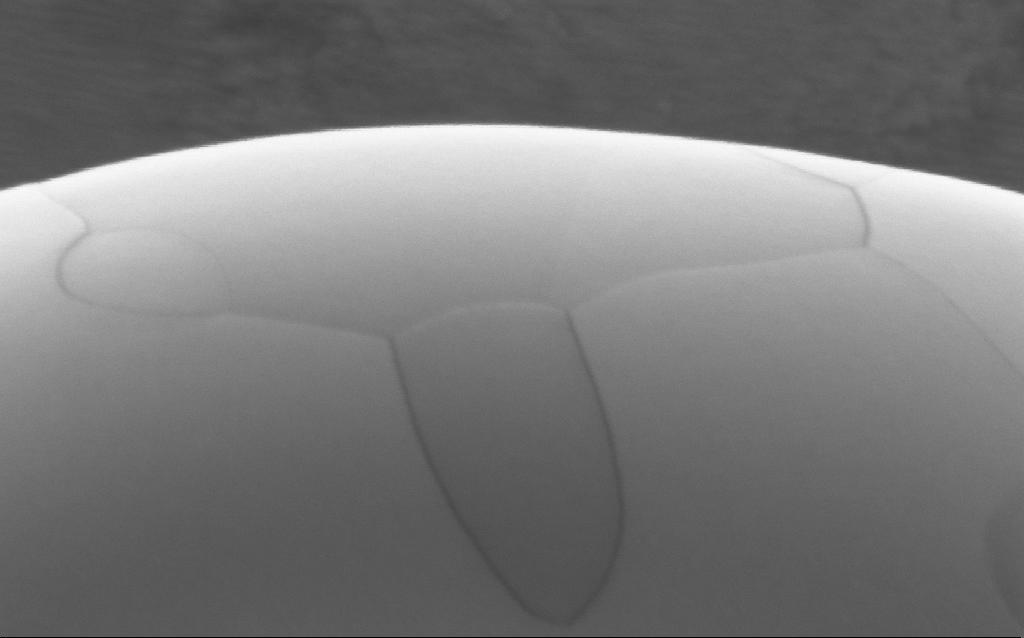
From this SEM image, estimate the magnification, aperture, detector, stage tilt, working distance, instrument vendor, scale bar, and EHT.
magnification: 301.69 K X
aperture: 30 µm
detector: InLens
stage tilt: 0°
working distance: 4 mm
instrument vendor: Zeiss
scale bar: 100 nm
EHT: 5 kV